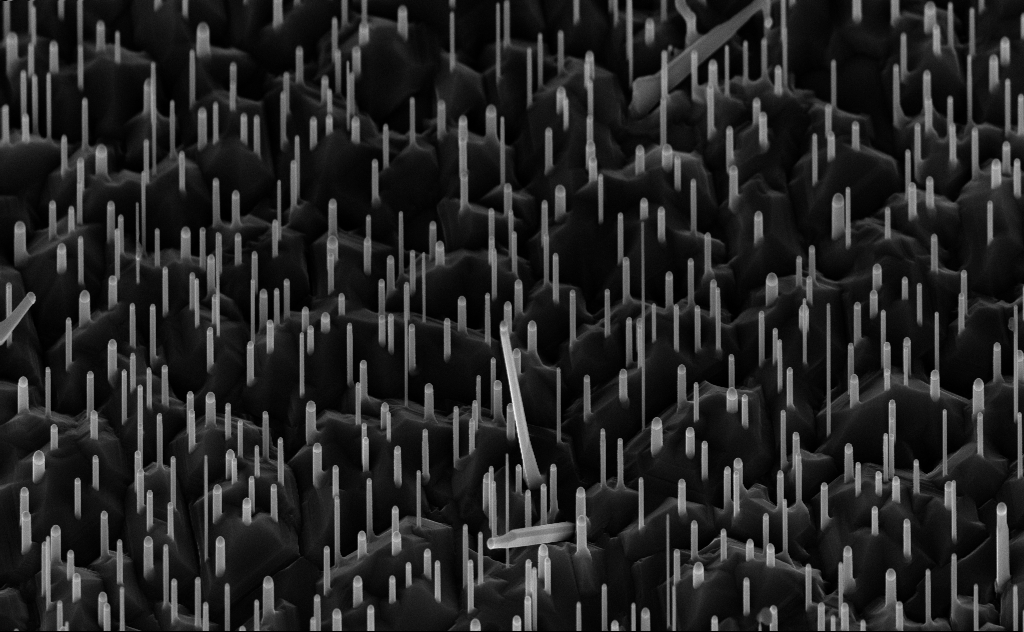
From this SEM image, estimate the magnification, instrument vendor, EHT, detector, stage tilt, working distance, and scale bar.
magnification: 25.04 K X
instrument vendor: Zeiss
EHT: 10 kV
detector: InLens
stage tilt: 45°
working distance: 7 mm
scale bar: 2000 nm